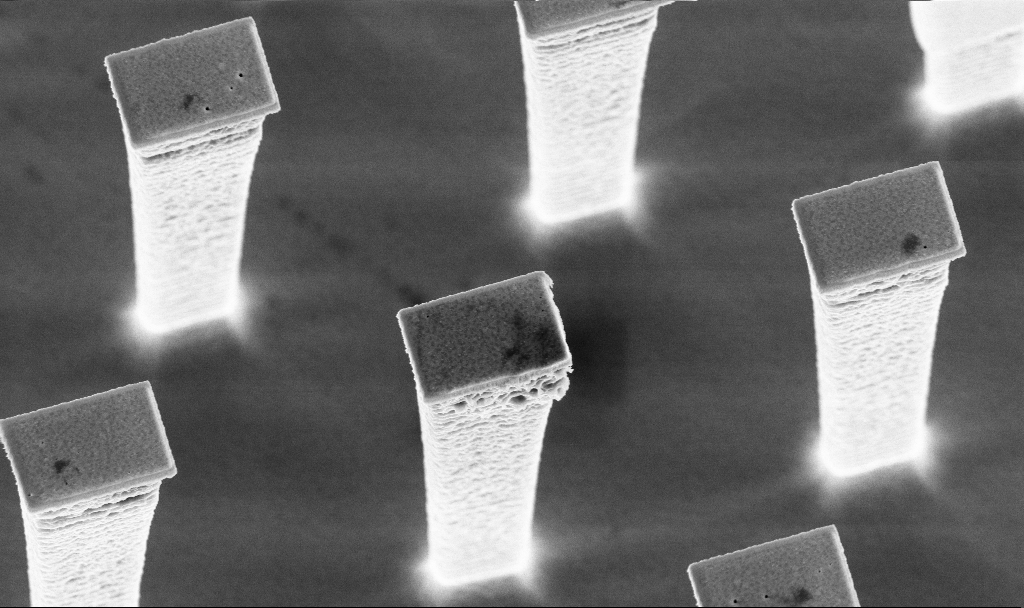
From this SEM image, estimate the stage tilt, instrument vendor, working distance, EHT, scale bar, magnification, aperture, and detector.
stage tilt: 20°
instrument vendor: Zeiss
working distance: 3 mm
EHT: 5 kV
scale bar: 2000 nm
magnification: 12.64 K X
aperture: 30 µm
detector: InLens